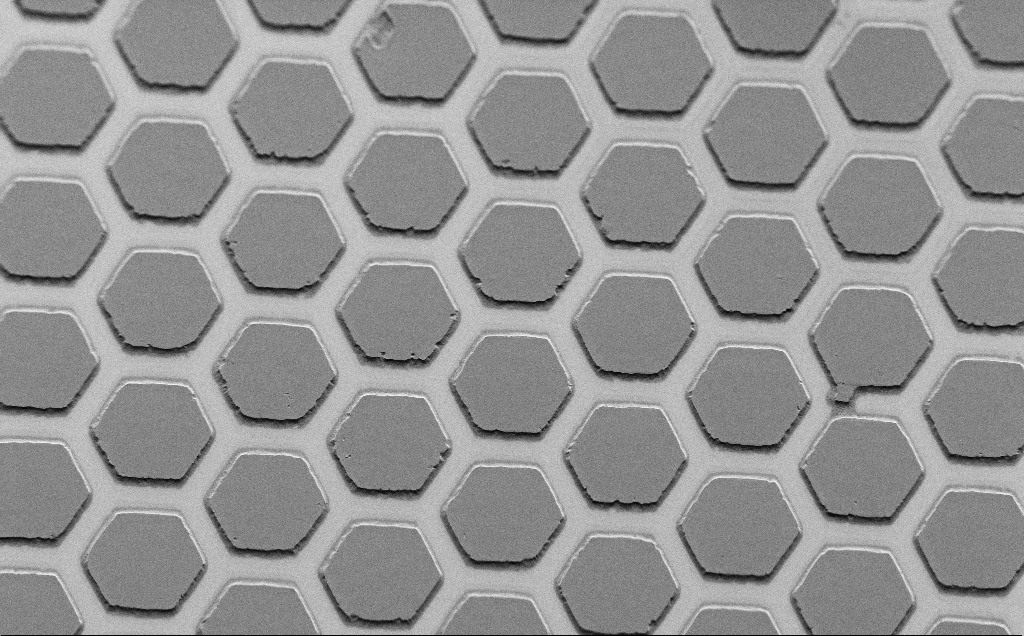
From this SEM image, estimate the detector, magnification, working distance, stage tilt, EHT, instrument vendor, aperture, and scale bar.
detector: SE2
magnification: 0.832 K X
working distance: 7 mm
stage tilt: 45°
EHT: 1.5 kV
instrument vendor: Zeiss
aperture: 30 µm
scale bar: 20000 nm